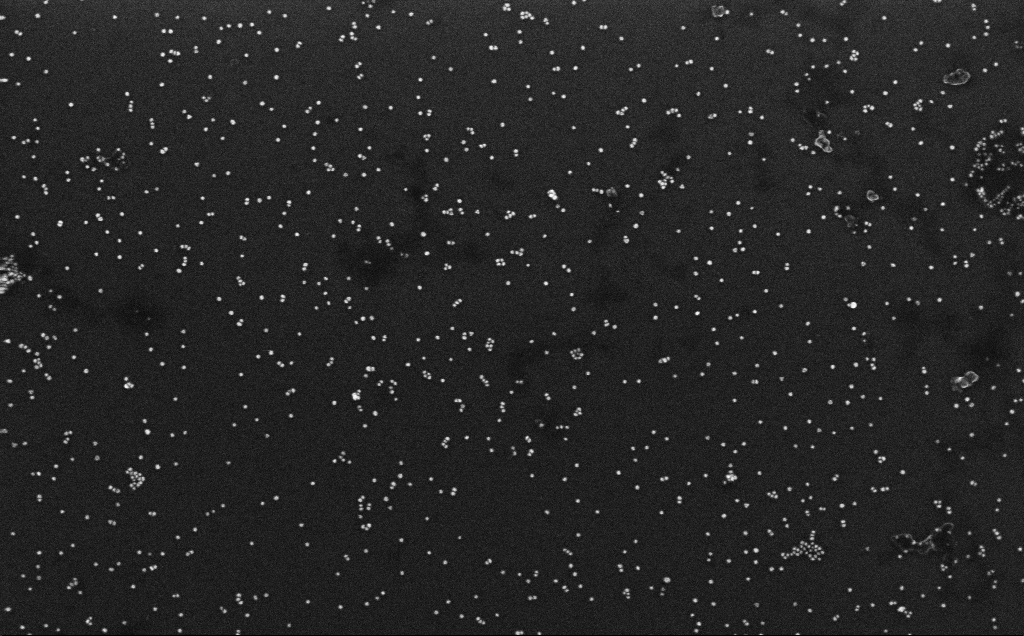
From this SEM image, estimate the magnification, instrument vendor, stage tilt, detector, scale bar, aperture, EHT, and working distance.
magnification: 100 K X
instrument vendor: Zeiss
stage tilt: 0°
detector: InLens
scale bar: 200 nm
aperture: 30 µm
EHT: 10 kV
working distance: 3.3 mm